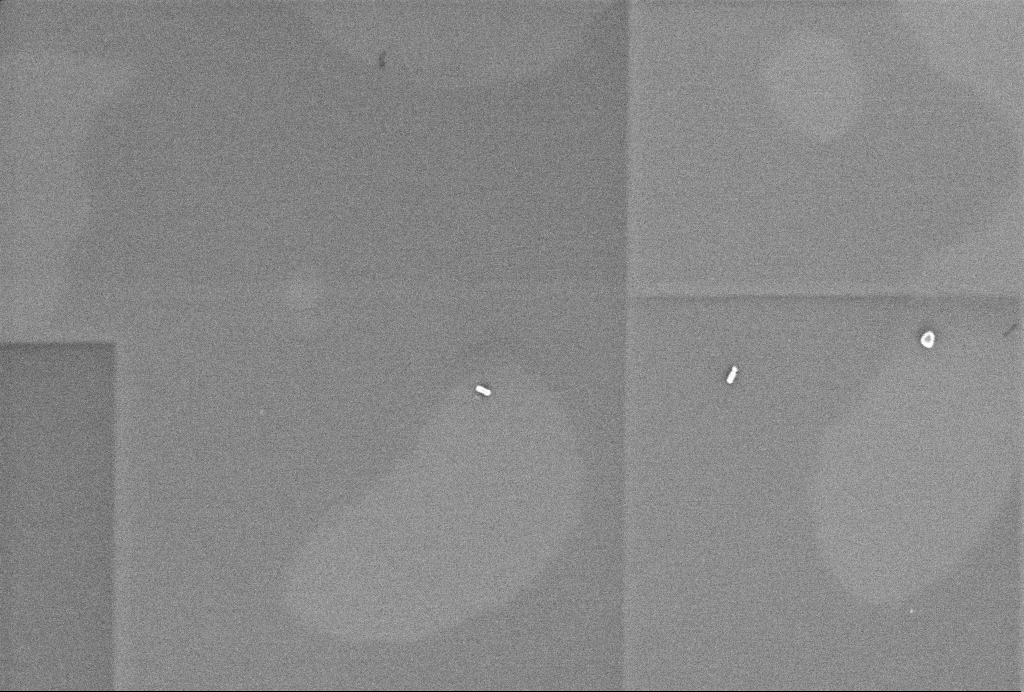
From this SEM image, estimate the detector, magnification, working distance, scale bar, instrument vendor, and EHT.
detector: InLens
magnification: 65 K X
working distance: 3.3 mm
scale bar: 200 nm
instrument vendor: Zeiss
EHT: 1 kV